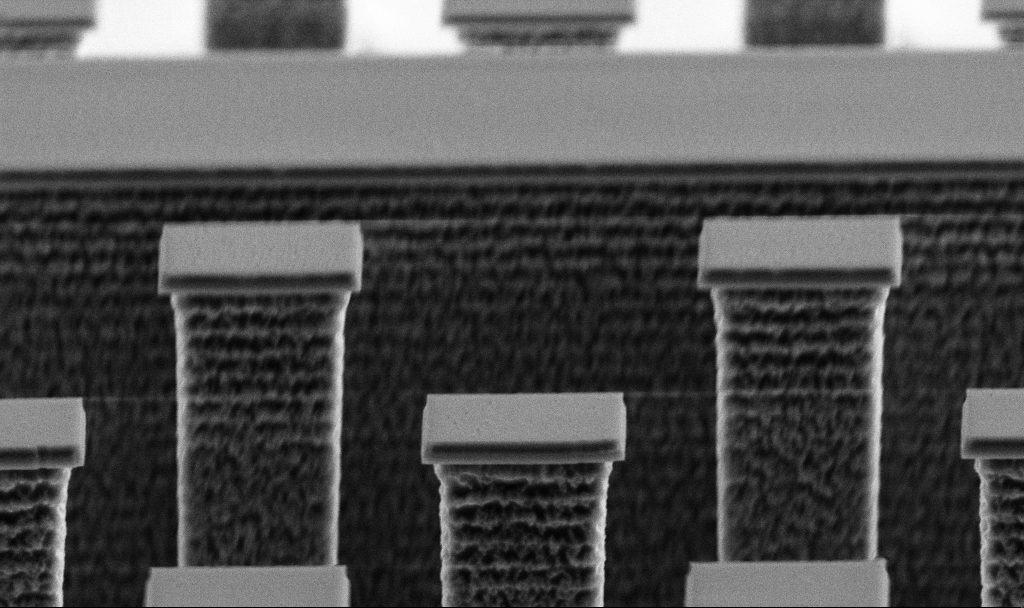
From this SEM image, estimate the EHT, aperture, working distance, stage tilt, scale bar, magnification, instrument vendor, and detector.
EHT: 5 kV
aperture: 30 µm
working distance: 5.5 mm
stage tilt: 70°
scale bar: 1000 nm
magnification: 17.13 K X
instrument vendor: Zeiss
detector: SE2